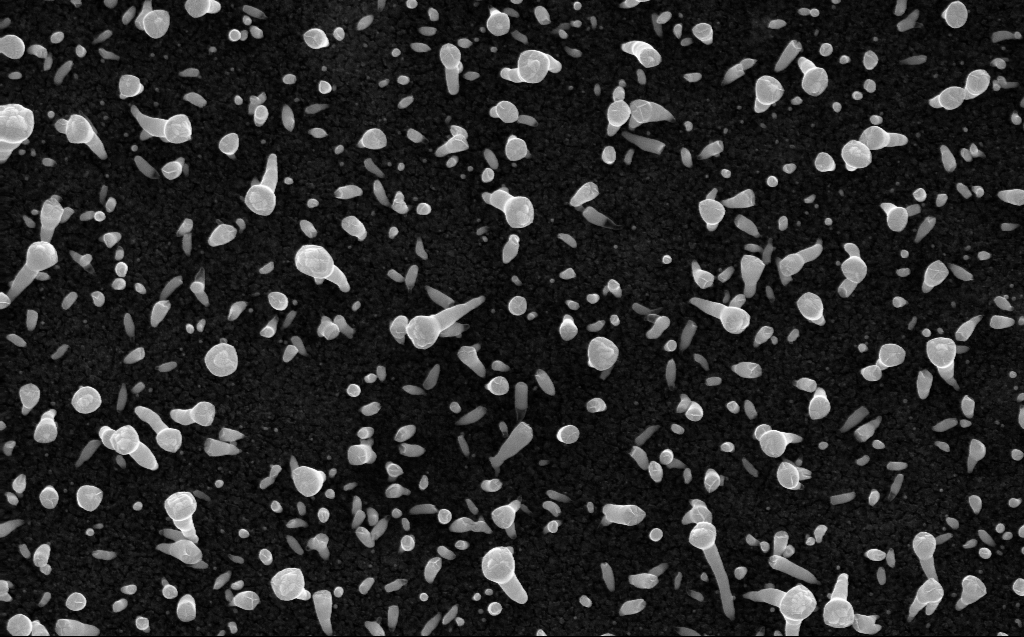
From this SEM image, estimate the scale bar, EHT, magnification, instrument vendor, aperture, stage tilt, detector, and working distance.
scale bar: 1000 nm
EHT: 10 kV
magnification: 50 K X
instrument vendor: Zeiss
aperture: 30 µm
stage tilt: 0°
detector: InLens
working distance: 3 mm